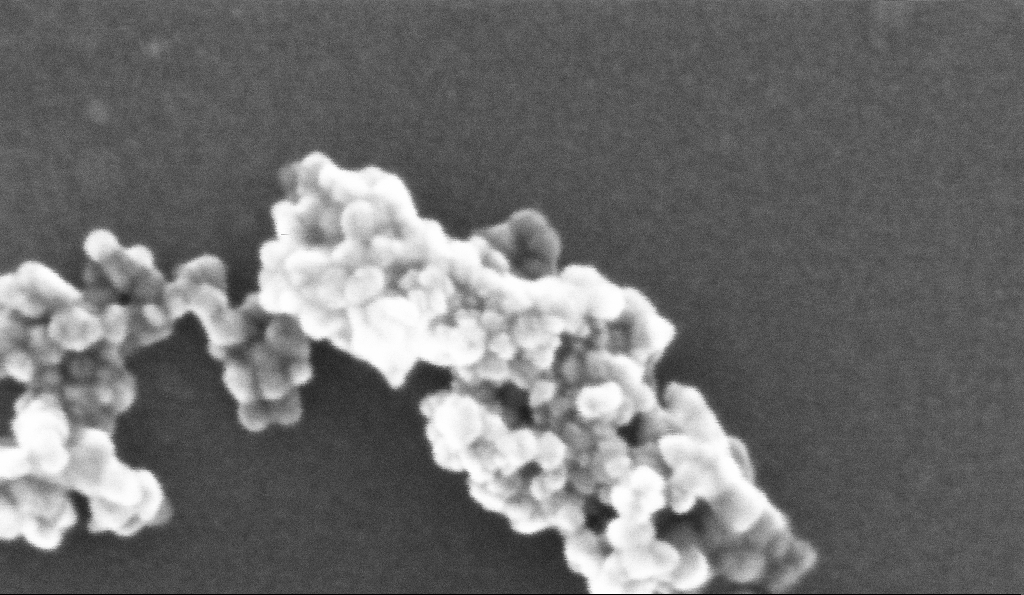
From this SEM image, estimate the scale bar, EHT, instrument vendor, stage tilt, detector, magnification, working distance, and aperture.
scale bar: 20 nm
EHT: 10 kV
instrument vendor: Zeiss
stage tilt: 0°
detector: InLens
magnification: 739.83 K X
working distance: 5.2 mm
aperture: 30 µm